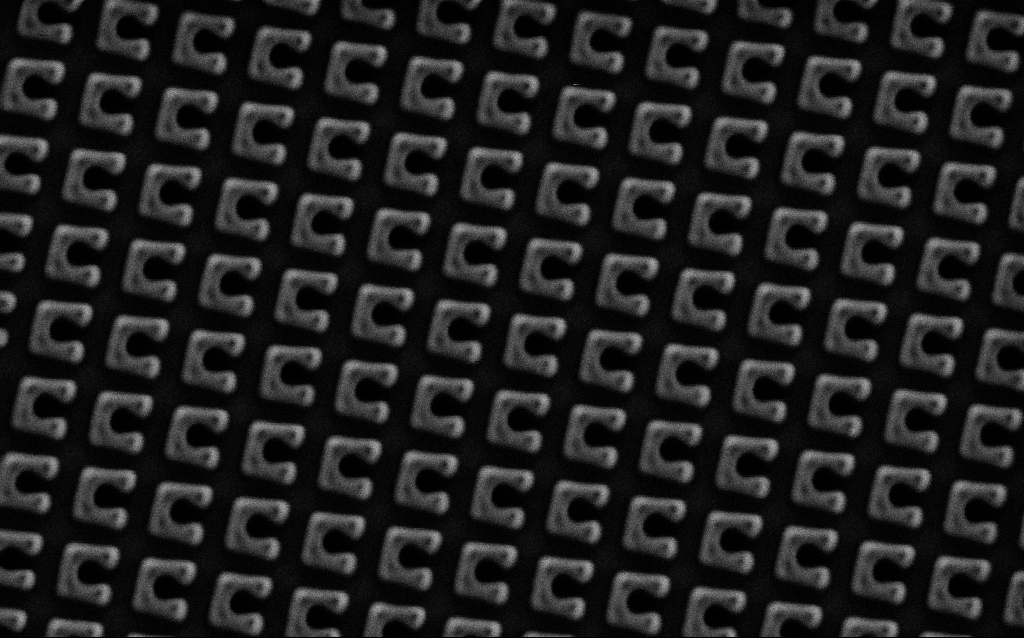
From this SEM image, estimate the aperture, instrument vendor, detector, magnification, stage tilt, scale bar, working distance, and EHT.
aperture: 30 µm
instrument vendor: Zeiss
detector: SE2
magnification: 61.41 K X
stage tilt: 0°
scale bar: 1000 nm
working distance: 7.7 mm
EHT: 1.5 kV